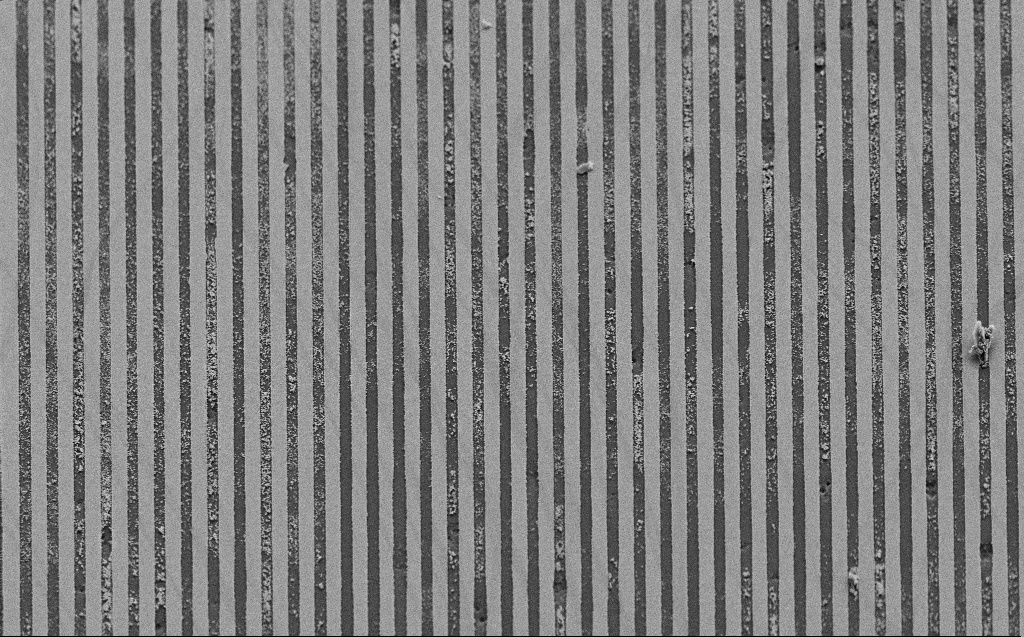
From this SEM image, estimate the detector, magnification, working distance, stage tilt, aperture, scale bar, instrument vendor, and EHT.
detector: SE2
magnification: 1.23 K X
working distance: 8 mm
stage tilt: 45°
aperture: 30 µm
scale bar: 20000 nm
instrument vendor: Zeiss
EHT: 10 kV